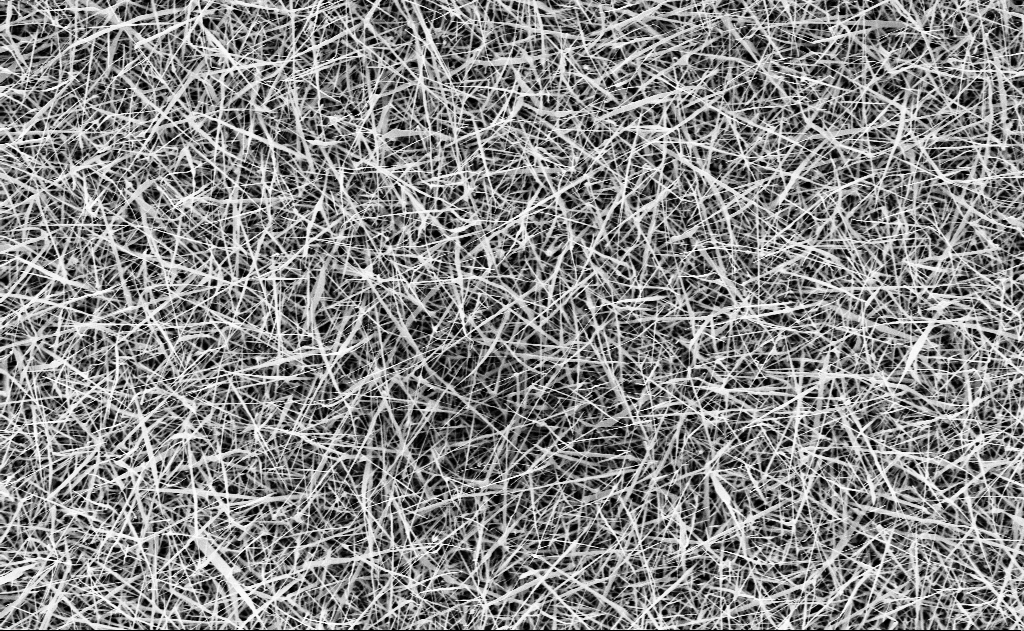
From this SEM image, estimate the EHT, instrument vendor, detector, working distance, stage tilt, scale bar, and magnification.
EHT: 10 kV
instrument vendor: Zeiss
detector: InLens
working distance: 15 mm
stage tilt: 0°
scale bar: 2000 nm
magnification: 10 K X